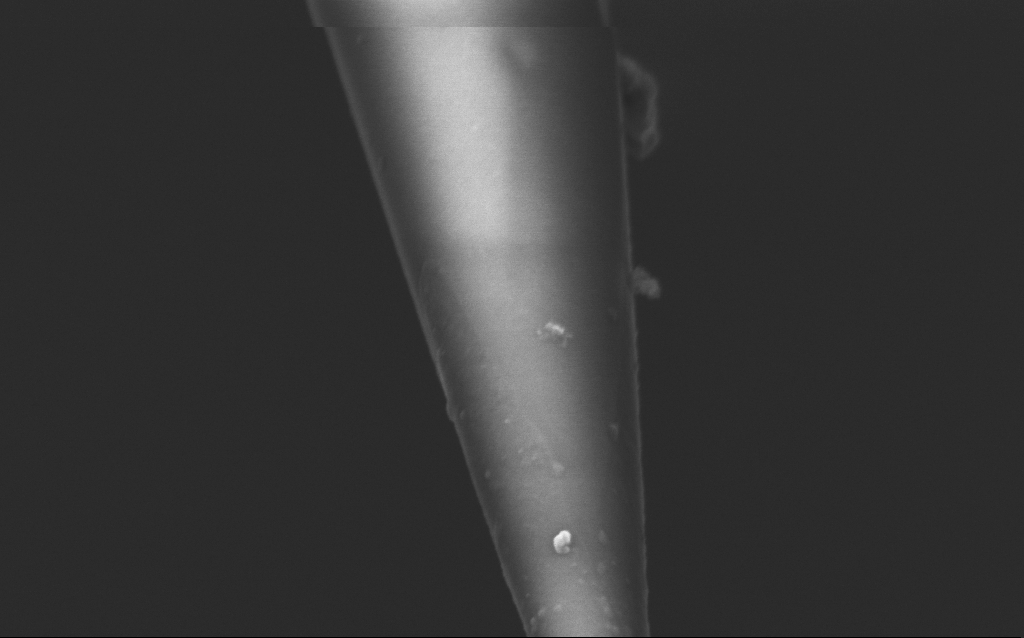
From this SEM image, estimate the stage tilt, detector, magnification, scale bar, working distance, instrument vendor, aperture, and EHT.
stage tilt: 45°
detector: InLens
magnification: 100 K X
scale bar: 200 nm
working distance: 6 mm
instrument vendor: Zeiss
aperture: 30 µm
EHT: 2.5 kV